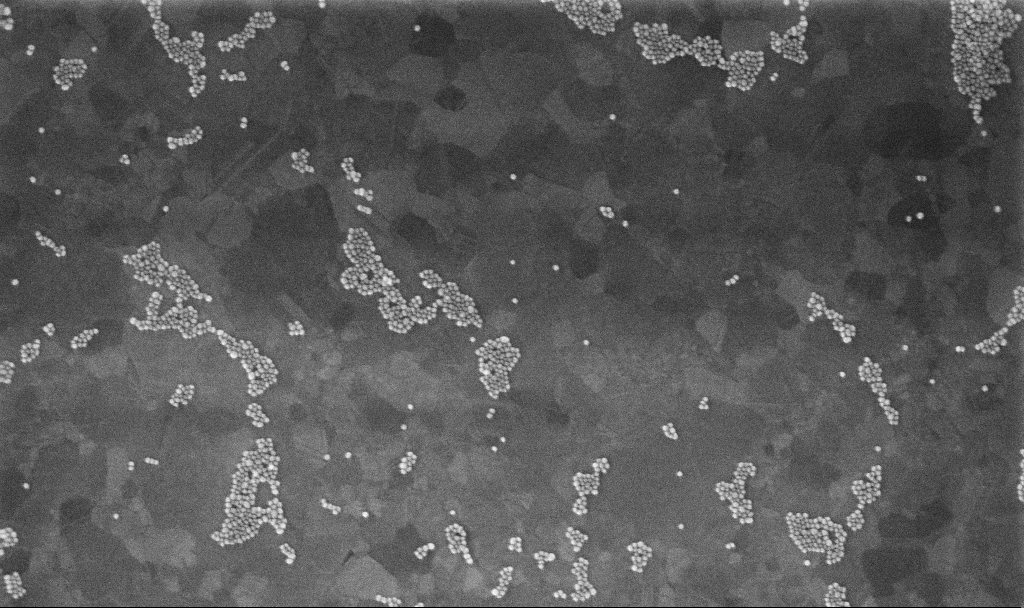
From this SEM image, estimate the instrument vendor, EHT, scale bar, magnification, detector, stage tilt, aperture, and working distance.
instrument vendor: Zeiss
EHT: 10 kV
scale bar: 200 nm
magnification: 100 K X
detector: InLens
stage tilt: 0°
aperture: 30 µm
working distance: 3.4 mm